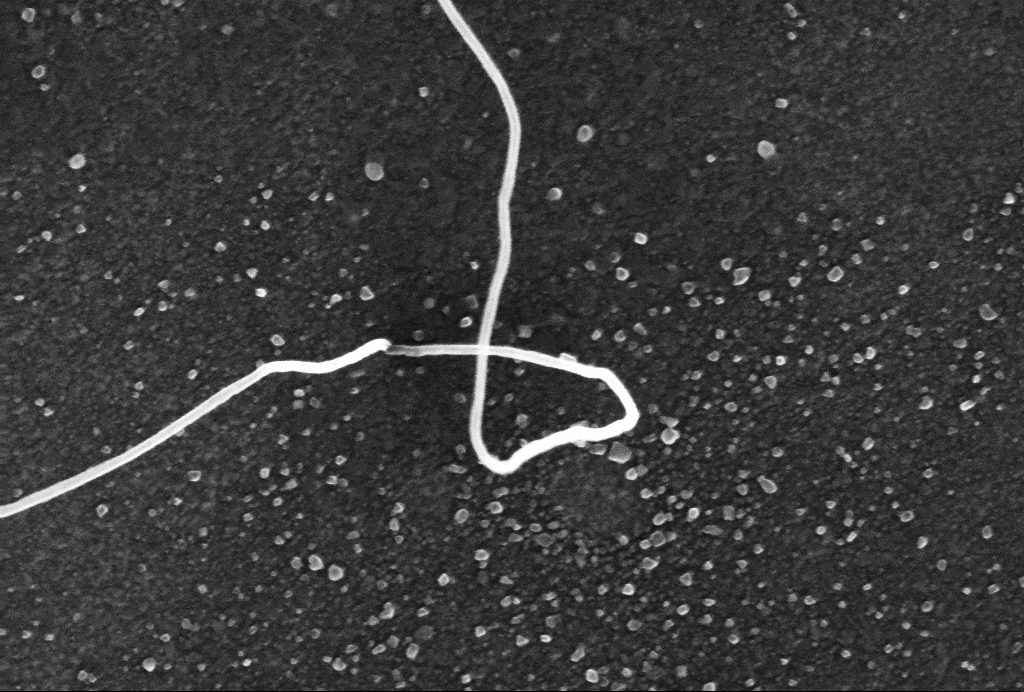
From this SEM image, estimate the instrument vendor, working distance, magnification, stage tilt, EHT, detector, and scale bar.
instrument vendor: Zeiss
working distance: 3.1 mm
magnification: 160.89 K X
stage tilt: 0°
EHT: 10 kV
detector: InLens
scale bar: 200 nm